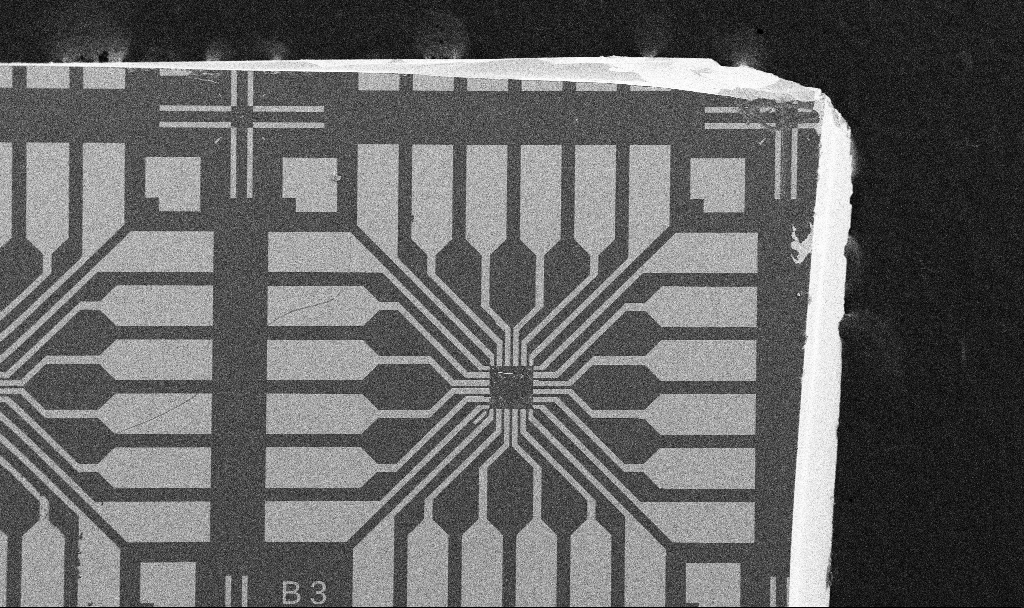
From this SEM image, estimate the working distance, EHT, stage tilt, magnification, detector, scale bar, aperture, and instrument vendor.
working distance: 10.7 mm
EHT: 10 kV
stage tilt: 0°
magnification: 0.1 K X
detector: SE2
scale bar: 200000 nm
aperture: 30 µm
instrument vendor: Zeiss